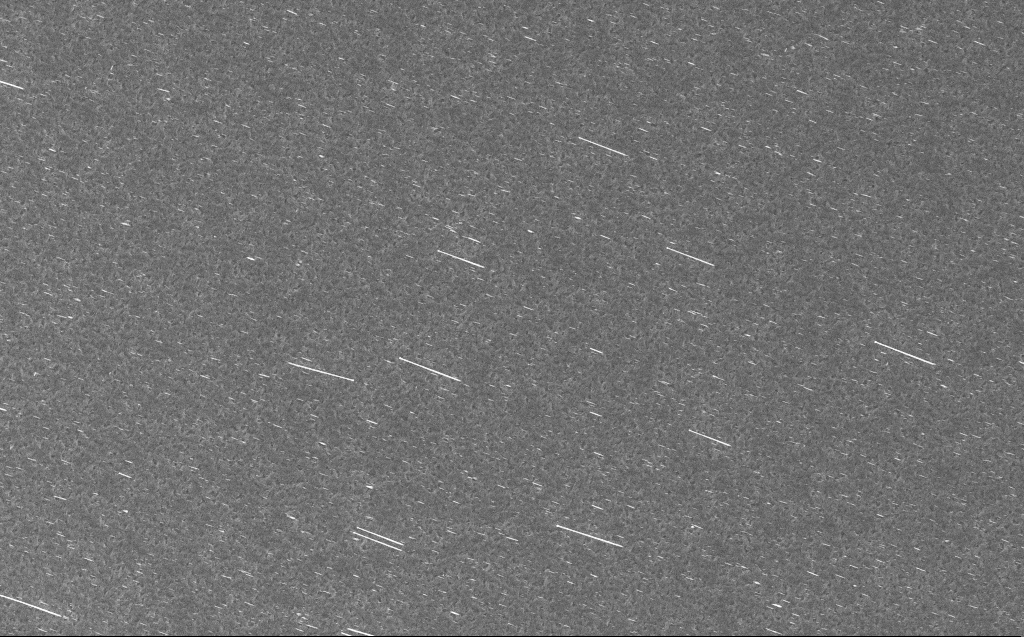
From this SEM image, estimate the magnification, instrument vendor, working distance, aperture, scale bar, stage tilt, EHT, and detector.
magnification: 5 K X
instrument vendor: Zeiss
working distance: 3 mm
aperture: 30 µm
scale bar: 10000 nm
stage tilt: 0°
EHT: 10 kV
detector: InLens